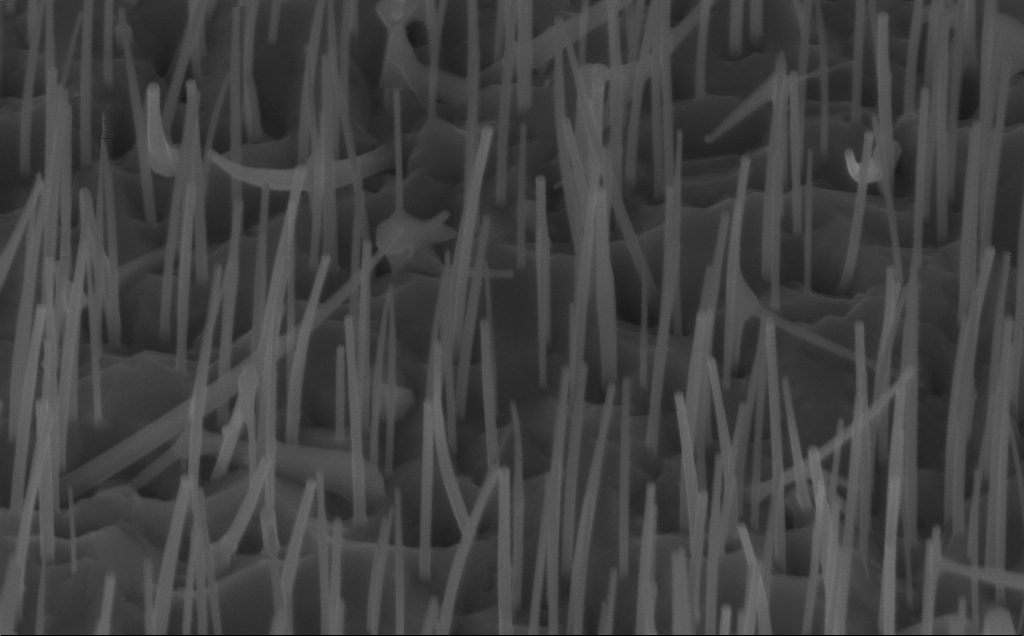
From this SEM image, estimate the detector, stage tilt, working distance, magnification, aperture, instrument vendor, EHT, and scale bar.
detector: InLens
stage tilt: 45°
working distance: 7 mm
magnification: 80 K X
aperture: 30 µm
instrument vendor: Zeiss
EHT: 10 kV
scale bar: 200 nm